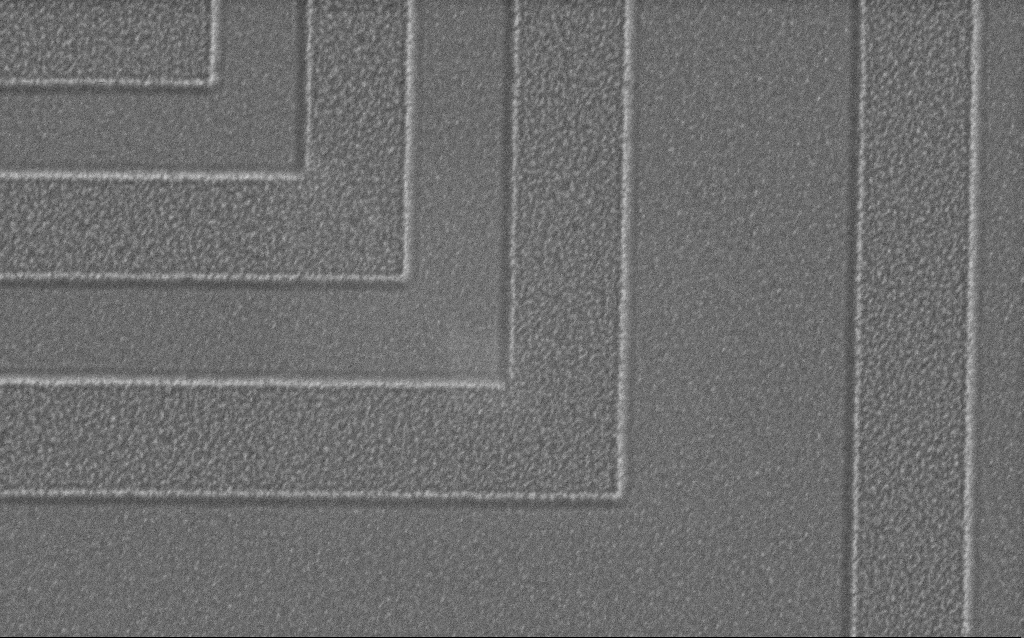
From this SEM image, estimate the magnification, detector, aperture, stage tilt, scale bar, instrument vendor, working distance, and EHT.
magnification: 42.04 K X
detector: SE2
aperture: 30 µm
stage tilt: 0°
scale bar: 1000 nm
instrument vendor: Zeiss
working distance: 6 mm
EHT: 1.5 kV